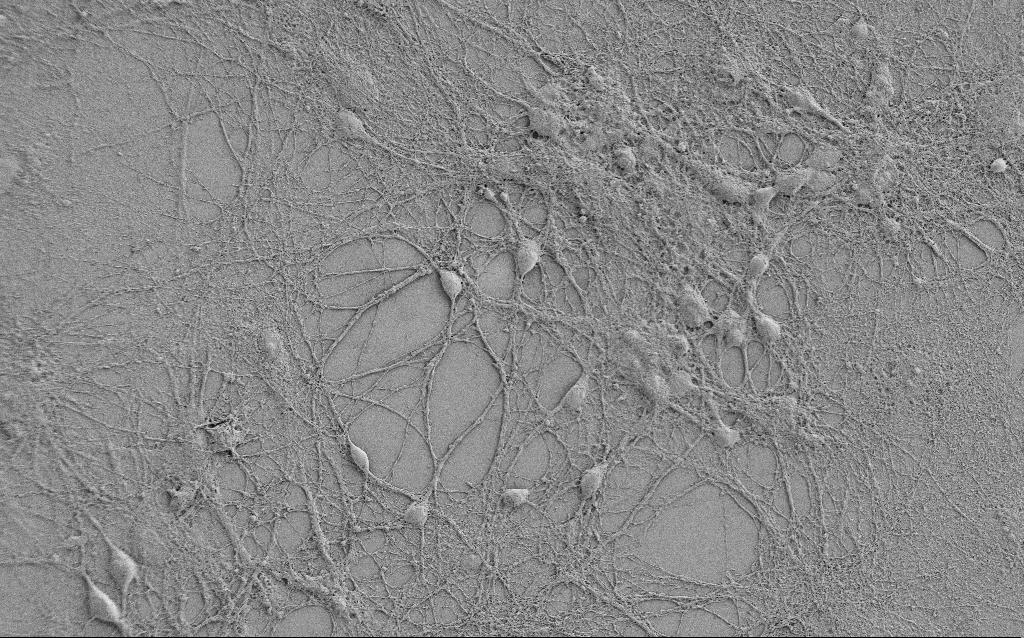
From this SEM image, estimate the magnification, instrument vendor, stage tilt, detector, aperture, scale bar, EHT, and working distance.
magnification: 0.75 K X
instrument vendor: Zeiss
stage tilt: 0°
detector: SE2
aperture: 30 µm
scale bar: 20000 nm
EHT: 0.8 kV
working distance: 5.8 mm